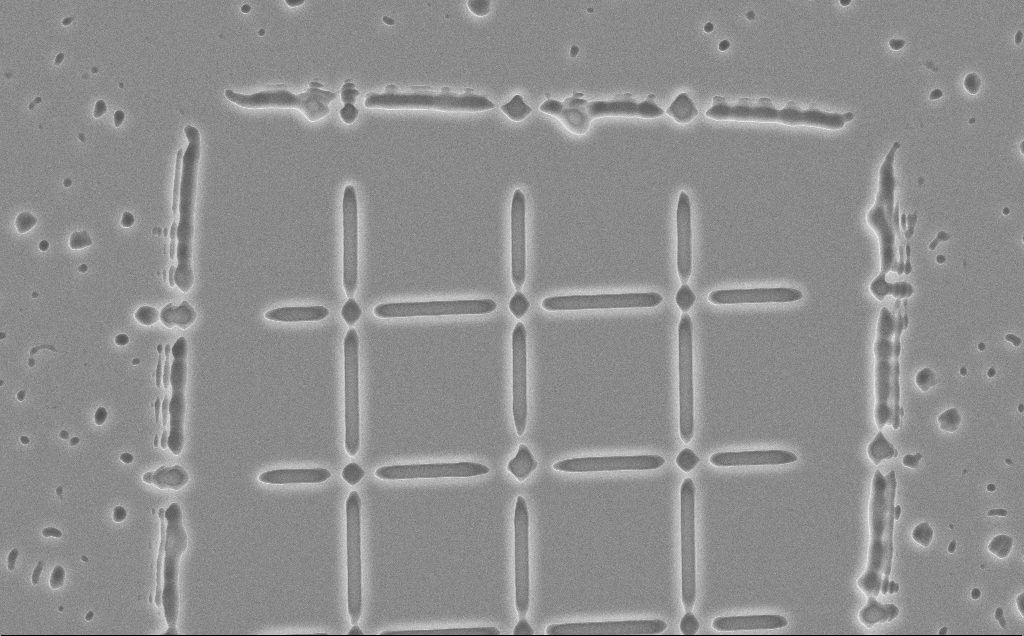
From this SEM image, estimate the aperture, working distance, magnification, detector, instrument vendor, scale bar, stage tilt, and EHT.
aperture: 30 µm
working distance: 13 mm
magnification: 3 K X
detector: SE2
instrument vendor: Zeiss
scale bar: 10000 nm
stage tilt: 0°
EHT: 10 kV